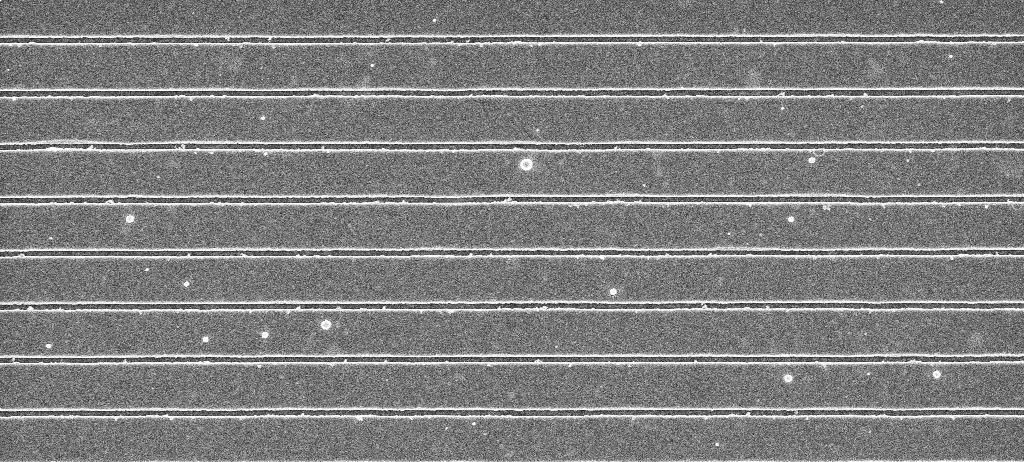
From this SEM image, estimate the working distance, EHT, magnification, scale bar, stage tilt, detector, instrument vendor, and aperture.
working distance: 5.3 mm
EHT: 5 kV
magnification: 21.85 K X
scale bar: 2000 nm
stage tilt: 0°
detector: InLens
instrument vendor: Zeiss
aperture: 30 µm